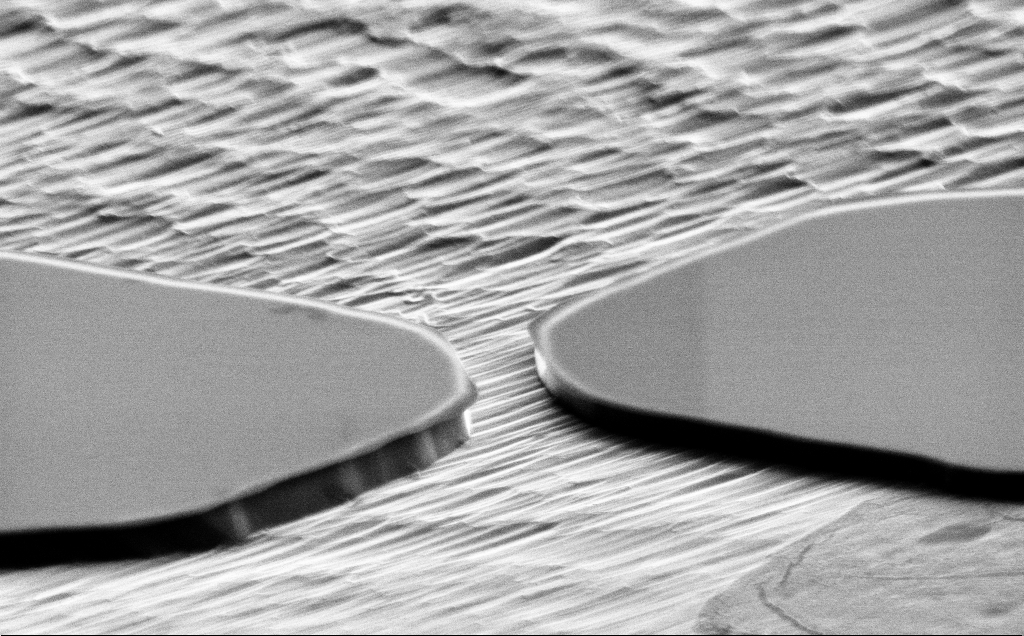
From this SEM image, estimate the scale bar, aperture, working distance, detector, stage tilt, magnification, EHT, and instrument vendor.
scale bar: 2000 nm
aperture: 30 µm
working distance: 10 mm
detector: SE2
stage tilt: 70°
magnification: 16.12 K X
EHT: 5 kV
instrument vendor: Zeiss